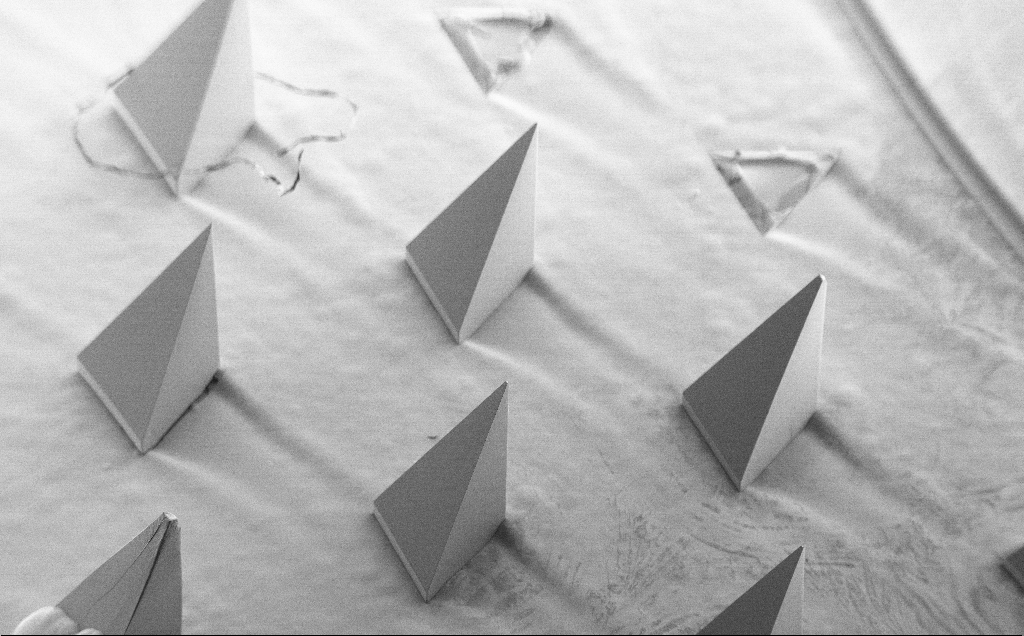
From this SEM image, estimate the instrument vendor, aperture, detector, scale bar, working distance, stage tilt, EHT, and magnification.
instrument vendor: Zeiss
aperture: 30 µm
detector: SE2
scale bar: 200000 nm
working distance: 8 mm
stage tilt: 40°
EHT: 5 kV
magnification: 0.081 K X